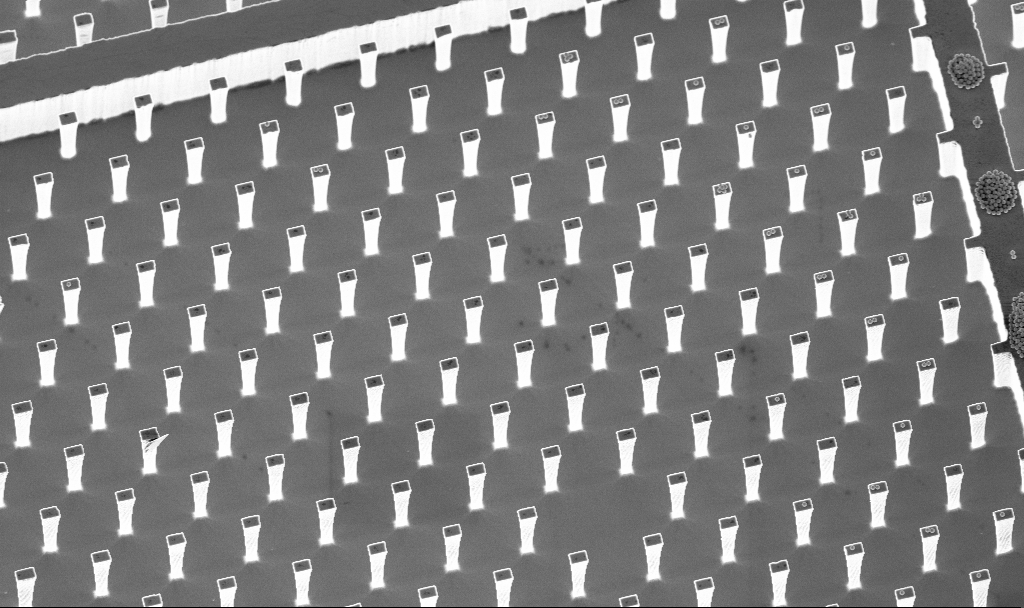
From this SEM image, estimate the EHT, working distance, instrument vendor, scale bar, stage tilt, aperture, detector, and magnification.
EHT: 5 kV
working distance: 4.7 mm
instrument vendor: Zeiss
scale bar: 20000 nm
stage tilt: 20°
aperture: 30 µm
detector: InLens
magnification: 2.37 K X